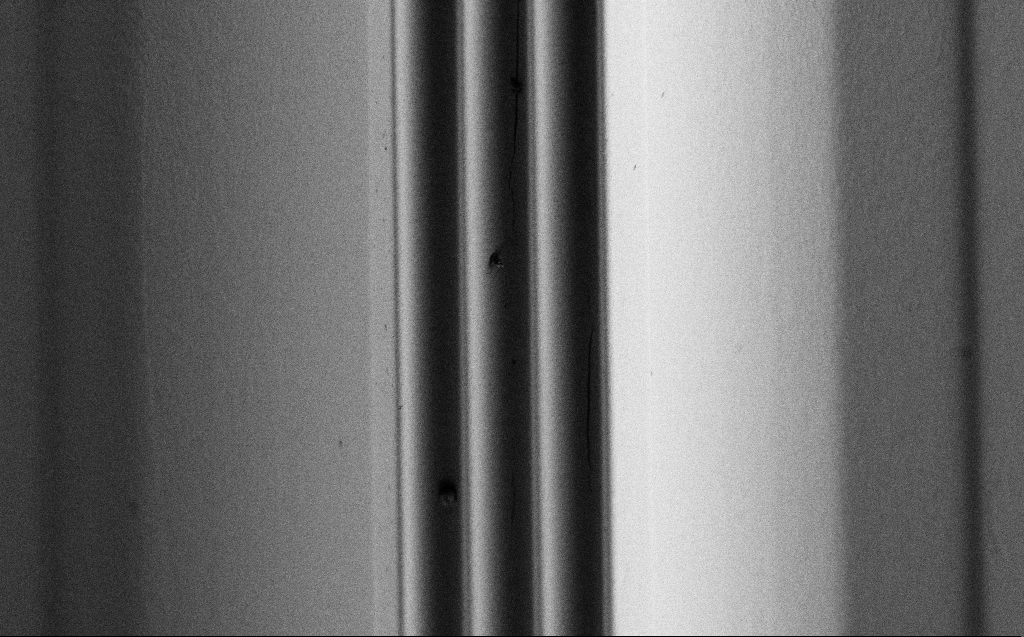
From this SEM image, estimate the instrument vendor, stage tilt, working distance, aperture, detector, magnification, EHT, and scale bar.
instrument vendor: Zeiss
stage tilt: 44.6°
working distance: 5 mm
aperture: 30 µm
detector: SE2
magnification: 2.27 K X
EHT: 1 kV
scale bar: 10000 nm